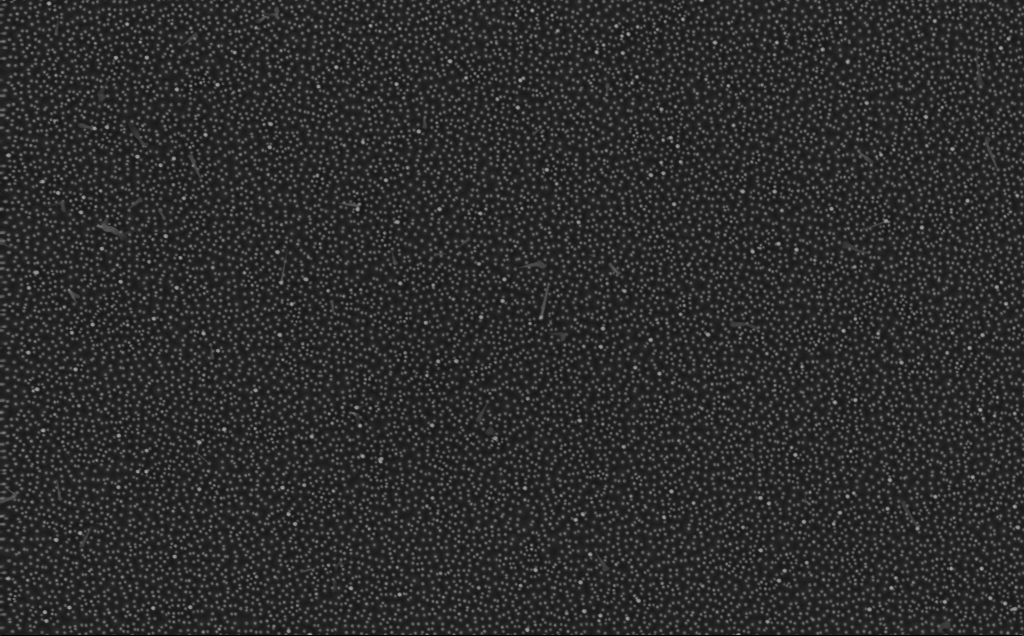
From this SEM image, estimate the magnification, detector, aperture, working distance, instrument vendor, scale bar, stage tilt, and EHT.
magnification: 20 K X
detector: InLens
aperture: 30 µm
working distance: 4 mm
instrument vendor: Zeiss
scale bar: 2000 nm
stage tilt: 0°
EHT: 10 kV